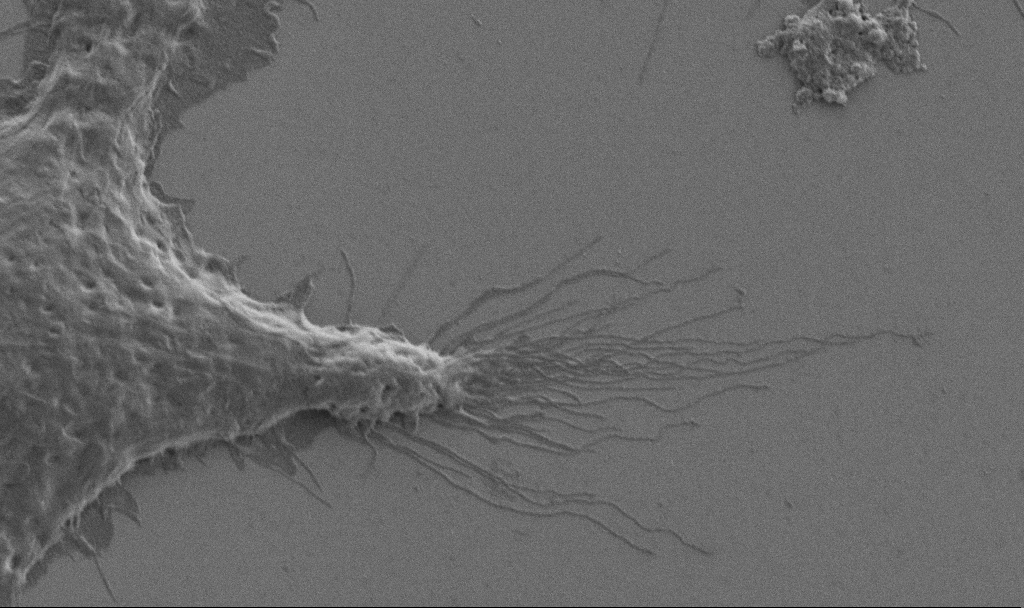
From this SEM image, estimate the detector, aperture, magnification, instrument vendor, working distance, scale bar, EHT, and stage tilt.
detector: SE2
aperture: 30 µm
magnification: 10 K X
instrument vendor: Zeiss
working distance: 6.9 mm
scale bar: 2000 nm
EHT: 1 kV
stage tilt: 0°